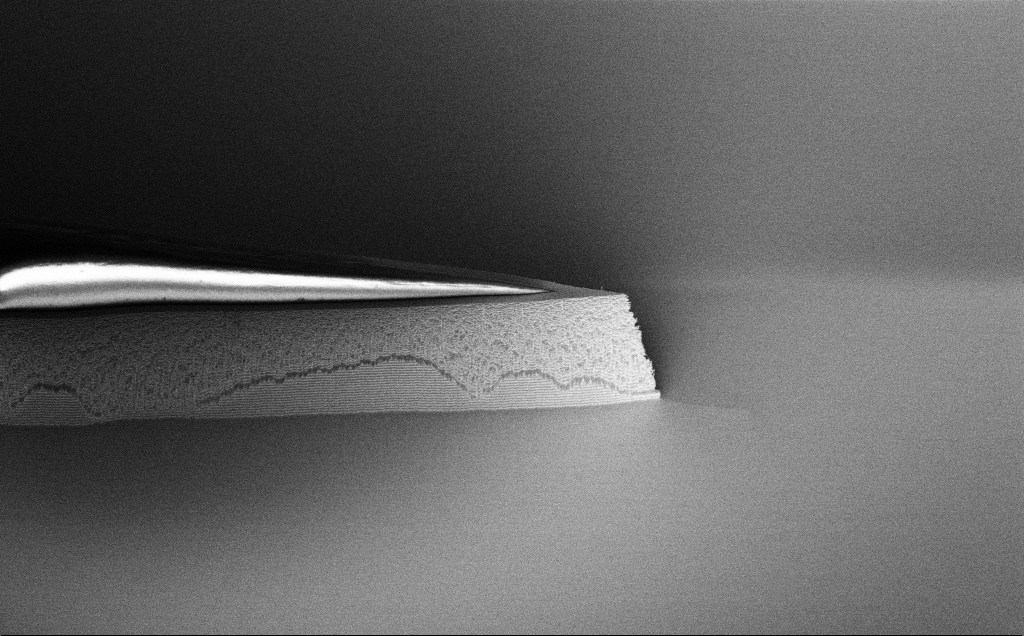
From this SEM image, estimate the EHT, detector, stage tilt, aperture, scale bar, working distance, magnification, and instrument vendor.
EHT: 1.2 kV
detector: InLens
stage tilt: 45°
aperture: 30 µm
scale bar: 20000 nm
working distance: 7 mm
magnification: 2.5 K X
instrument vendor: Zeiss